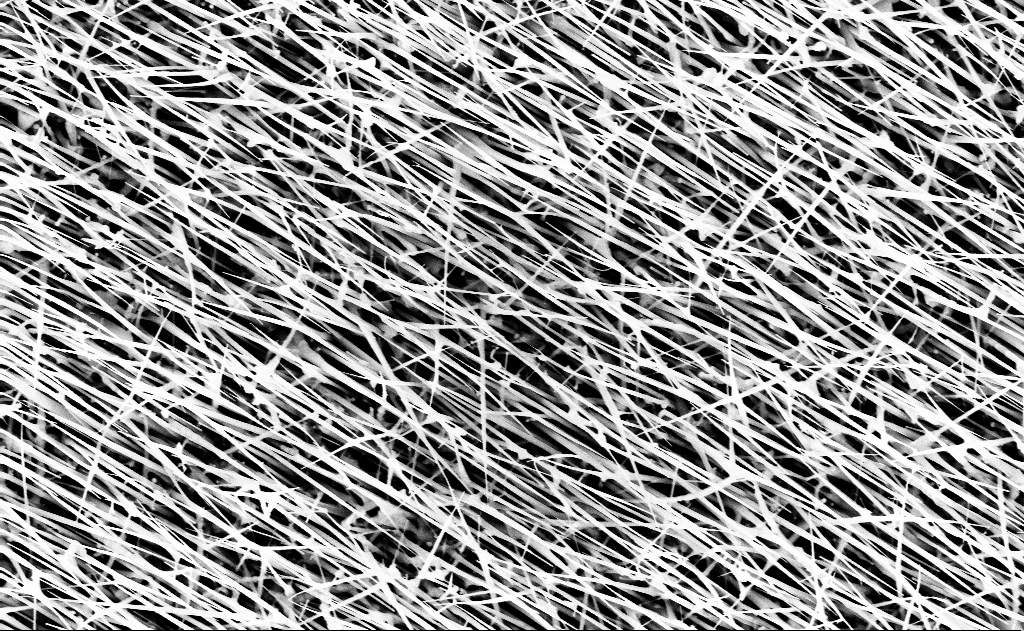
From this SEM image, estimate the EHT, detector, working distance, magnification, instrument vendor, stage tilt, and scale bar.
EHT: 10 kV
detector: InLens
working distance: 13 mm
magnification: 20 K X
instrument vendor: Zeiss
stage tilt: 0°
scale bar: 2000 nm